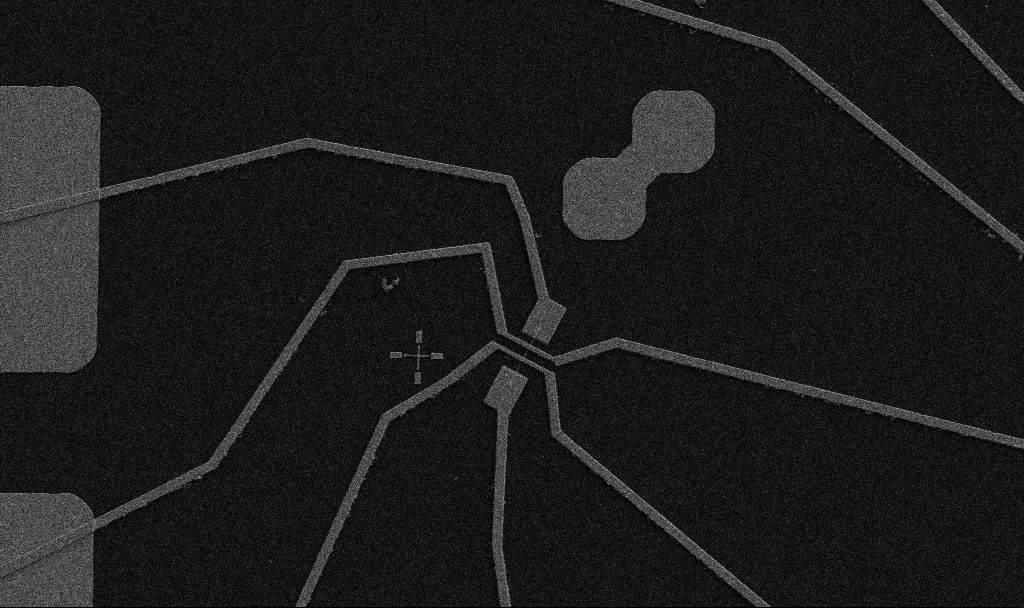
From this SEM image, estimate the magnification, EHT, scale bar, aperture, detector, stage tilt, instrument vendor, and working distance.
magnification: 5 K X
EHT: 5 kV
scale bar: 10000 nm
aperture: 30 µm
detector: SE2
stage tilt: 0°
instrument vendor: Zeiss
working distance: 10.7 mm